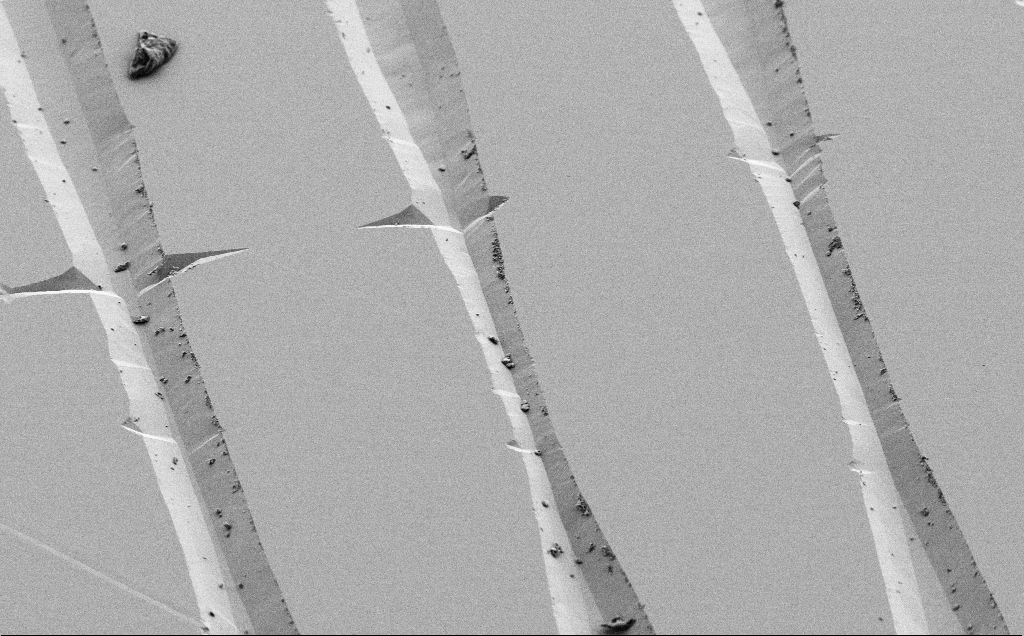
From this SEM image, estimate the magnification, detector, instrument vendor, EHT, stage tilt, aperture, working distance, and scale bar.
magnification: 1.17 K X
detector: SE2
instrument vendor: Zeiss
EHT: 3 kV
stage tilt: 45°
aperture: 30 µm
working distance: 9 mm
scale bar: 20000 nm